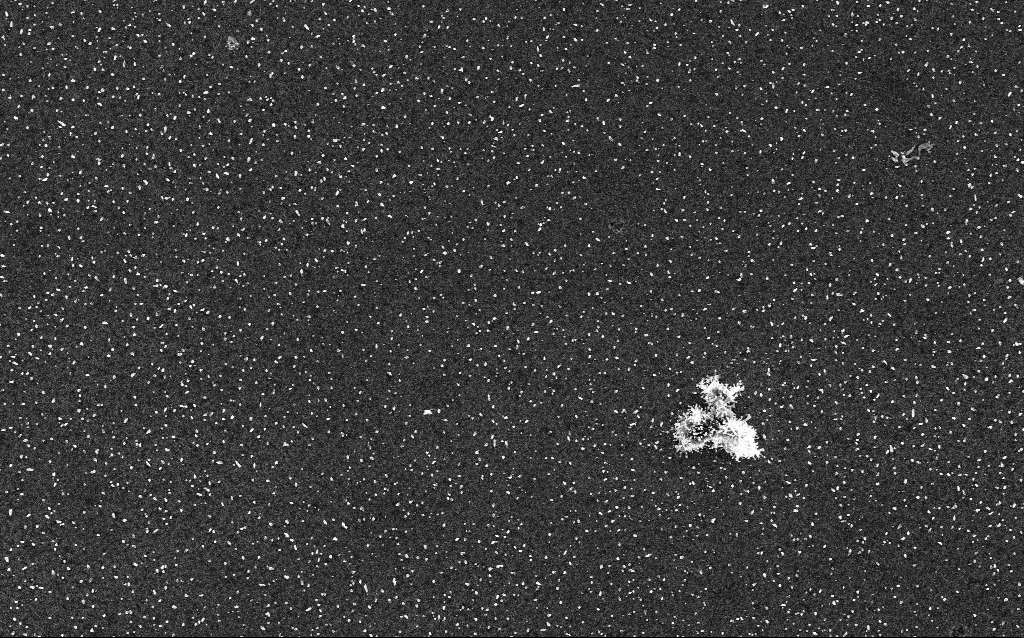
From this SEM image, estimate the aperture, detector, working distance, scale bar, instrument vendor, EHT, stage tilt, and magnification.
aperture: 30 µm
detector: InLens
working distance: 2.1 mm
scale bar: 2000 nm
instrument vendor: Zeiss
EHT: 5 kV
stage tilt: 0°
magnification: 10 K X